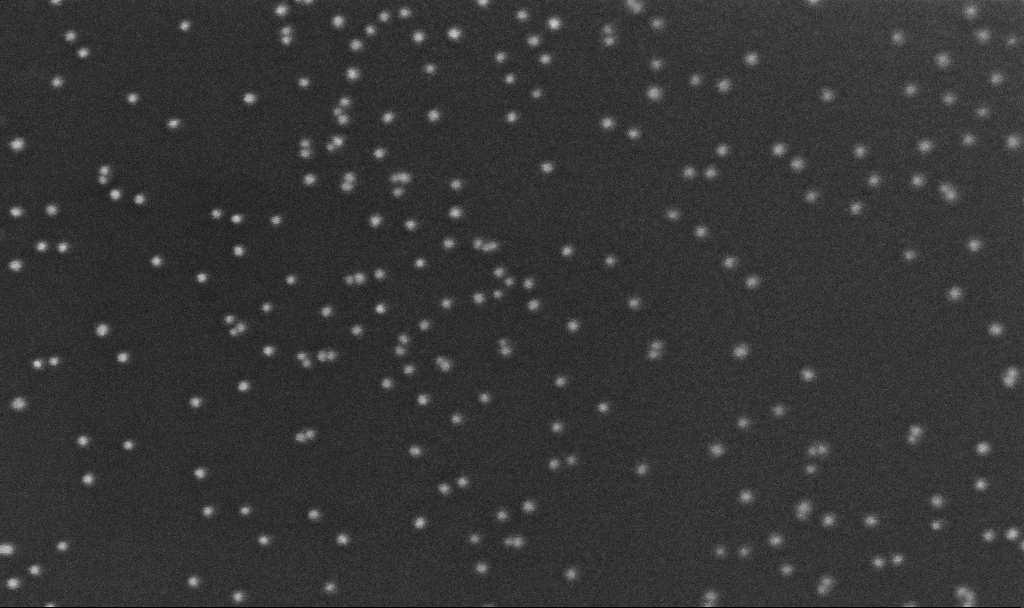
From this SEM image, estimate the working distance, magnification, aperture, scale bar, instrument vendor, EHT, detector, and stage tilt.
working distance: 3.2 mm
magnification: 350 K X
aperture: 30 µm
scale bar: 200 nm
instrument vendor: Zeiss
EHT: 10 kV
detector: InLens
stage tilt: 0°